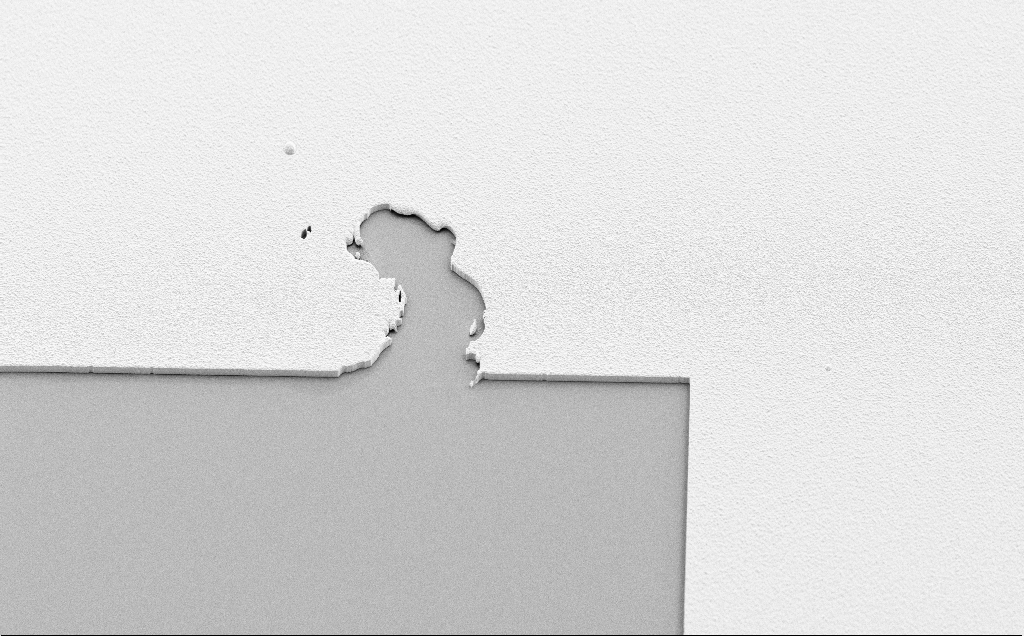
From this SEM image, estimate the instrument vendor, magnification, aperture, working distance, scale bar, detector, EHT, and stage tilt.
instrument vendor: Zeiss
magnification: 0.81 K X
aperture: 30 µm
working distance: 8 mm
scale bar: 20000 nm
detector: SE2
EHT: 10 kV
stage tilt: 45°